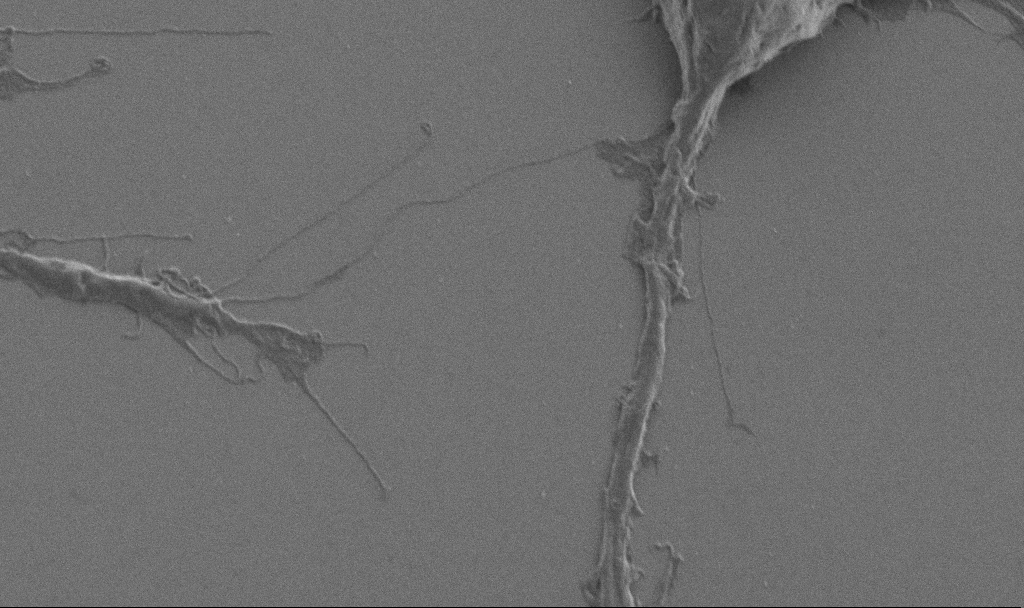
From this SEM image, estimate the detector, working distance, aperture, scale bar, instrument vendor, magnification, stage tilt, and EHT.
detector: SE2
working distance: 6.9 mm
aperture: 30 µm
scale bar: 2000 nm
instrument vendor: Zeiss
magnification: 10 K X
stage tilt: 0°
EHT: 1 kV